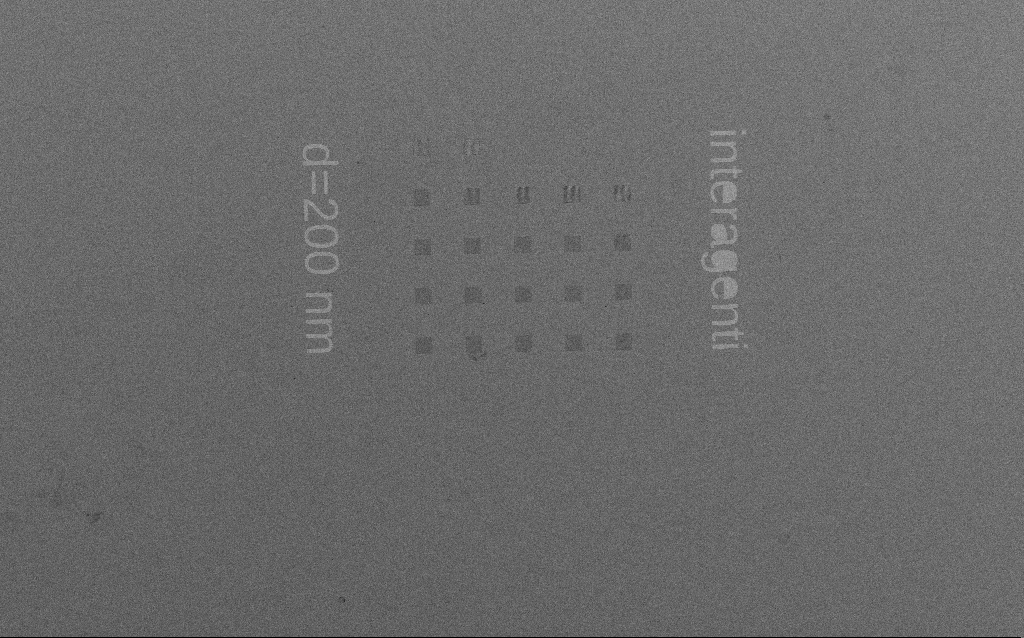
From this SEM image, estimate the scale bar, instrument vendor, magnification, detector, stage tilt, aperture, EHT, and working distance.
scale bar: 200000 nm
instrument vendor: Zeiss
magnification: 0.244 K X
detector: SE2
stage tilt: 0°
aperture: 30 µm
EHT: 1.5 kV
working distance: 5.8 mm